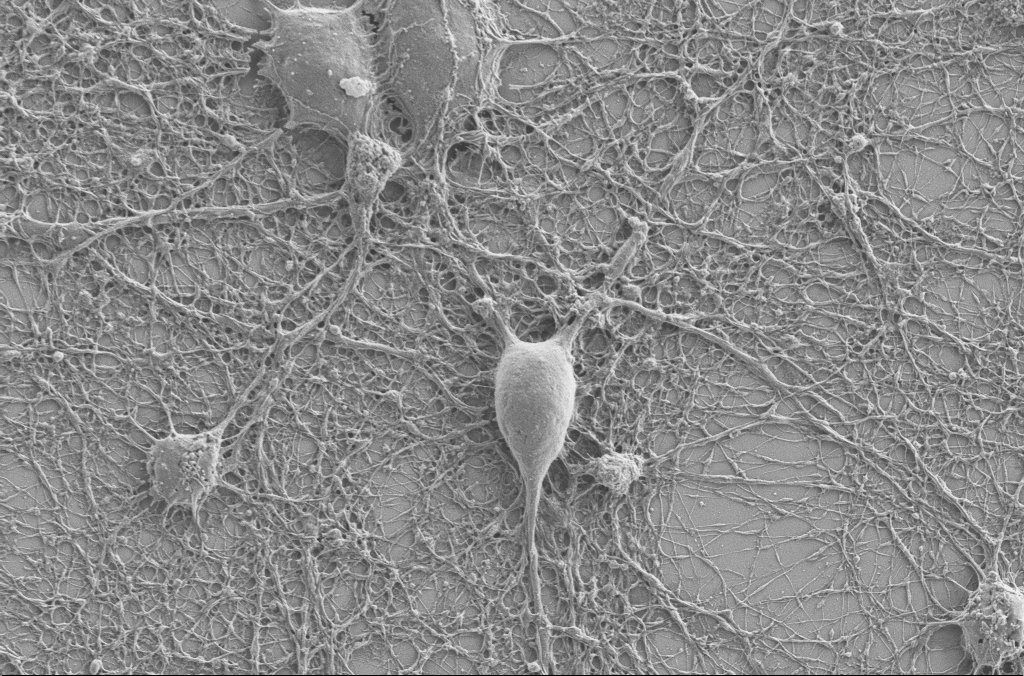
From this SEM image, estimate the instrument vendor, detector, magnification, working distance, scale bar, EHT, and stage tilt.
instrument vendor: Zeiss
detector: SE2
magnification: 5 K X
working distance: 4 mm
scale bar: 10000 nm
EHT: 2 kV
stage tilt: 0°